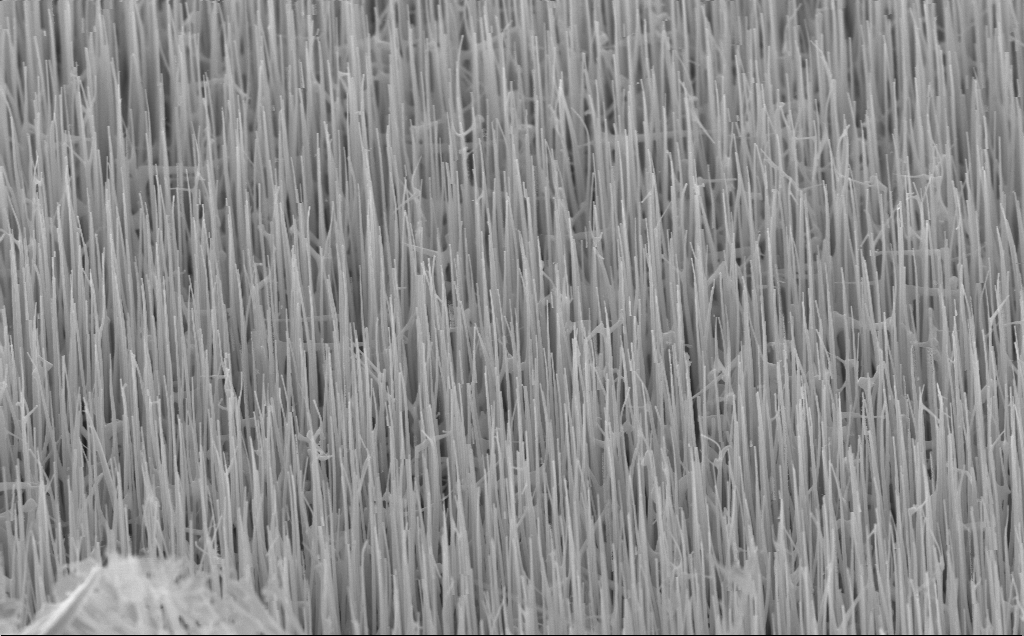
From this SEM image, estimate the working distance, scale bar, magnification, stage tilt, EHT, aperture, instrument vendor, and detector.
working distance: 7 mm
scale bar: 1000 nm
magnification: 29.82 K X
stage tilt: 45°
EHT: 10 kV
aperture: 30 µm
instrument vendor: Zeiss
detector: InLens